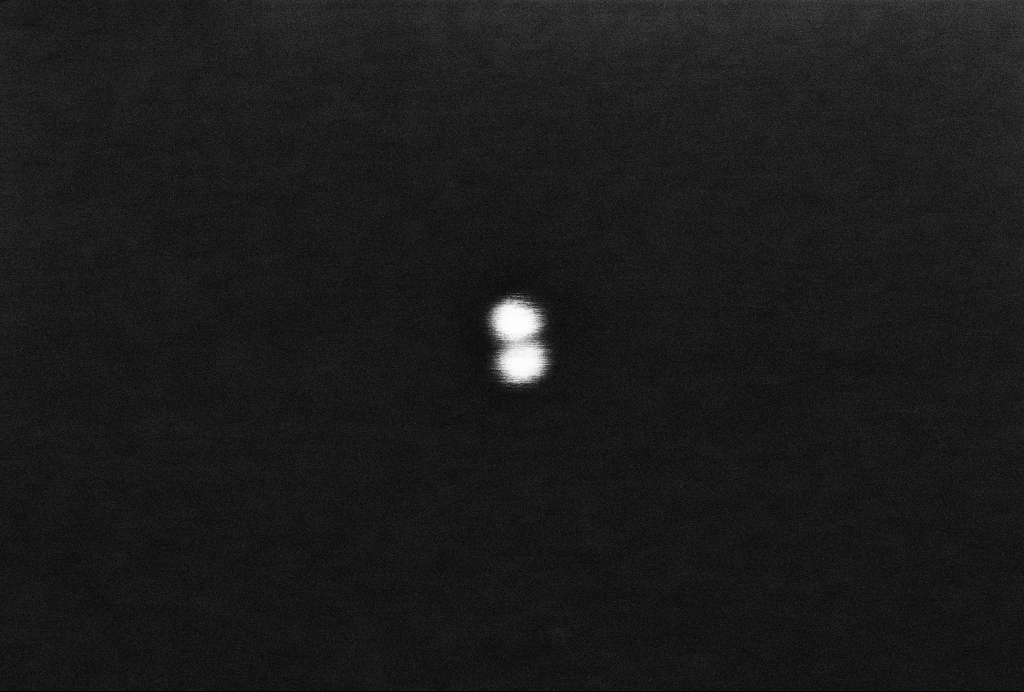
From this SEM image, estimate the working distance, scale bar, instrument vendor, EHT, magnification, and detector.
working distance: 3.3 mm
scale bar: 20 nm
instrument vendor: Zeiss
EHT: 2 kV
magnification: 669.09 K X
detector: InLens